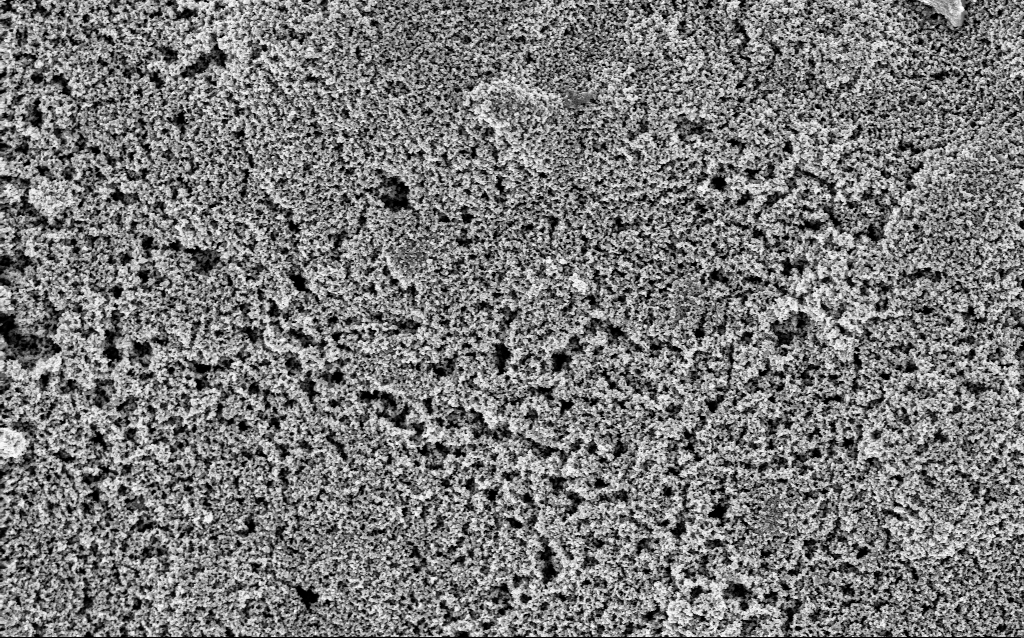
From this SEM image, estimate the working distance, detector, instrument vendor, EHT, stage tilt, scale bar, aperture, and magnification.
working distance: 7.5 mm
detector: InLens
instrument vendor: Zeiss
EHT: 3 kV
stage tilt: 0°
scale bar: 1000 nm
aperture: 30 µm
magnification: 23.9 K X